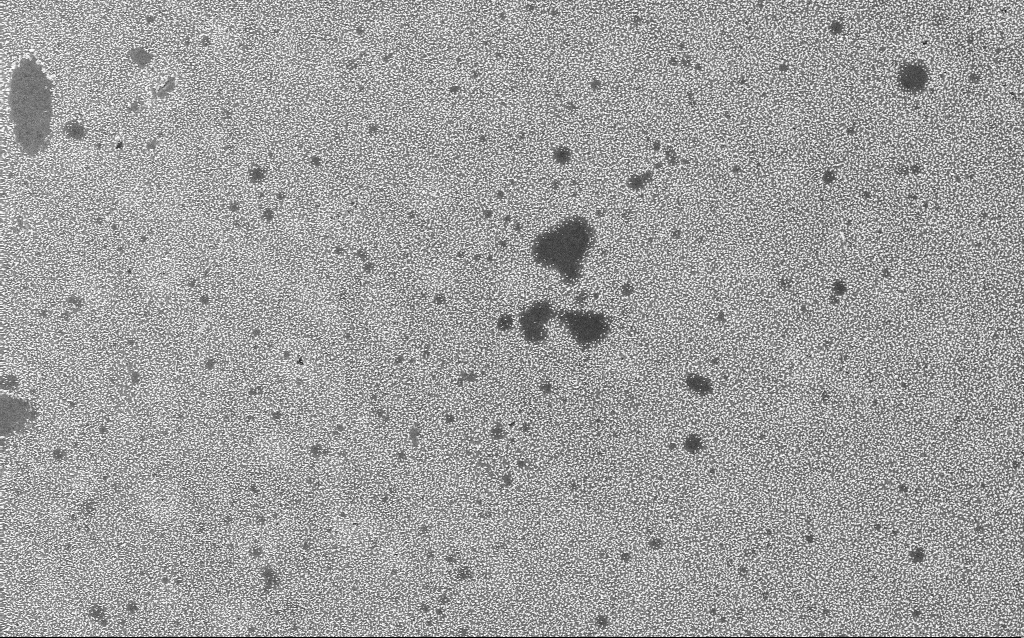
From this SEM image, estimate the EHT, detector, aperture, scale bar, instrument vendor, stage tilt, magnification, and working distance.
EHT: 8 kV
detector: InLens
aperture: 30 µm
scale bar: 10000 nm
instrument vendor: Zeiss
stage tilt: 0°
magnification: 2.6 K X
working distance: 5 mm